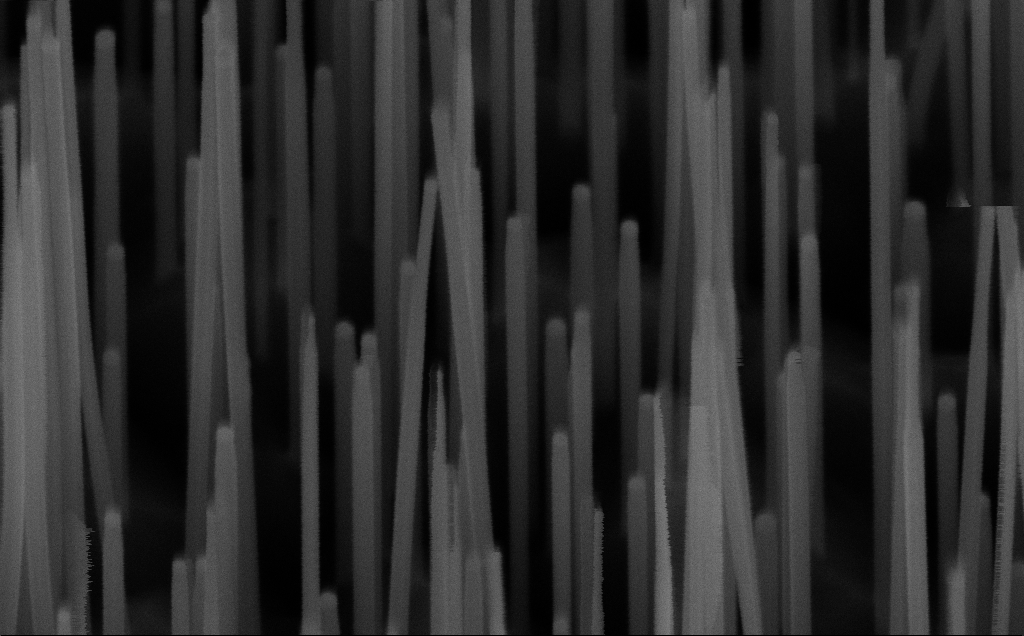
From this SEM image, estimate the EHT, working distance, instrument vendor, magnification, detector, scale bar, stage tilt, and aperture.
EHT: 10 kV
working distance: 7 mm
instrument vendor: Zeiss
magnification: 200 K X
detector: InLens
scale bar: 200 nm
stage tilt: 45°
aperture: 30 µm